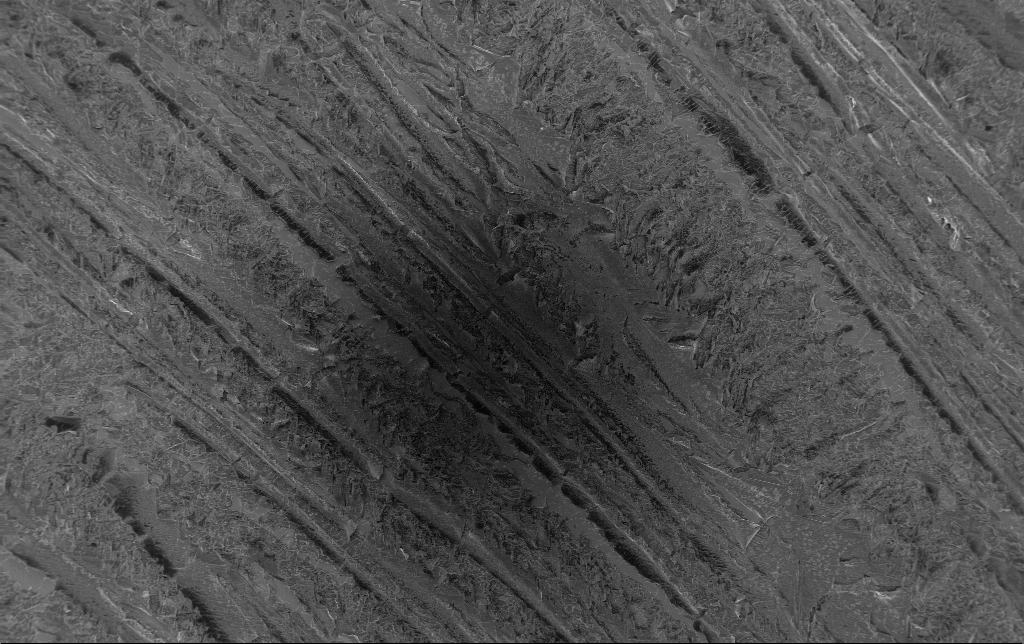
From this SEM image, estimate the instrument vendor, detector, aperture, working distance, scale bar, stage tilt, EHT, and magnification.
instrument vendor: Zeiss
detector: InLens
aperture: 30 µm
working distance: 3.2 mm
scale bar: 100000 nm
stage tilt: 0°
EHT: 1.5 kV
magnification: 0.271 K X